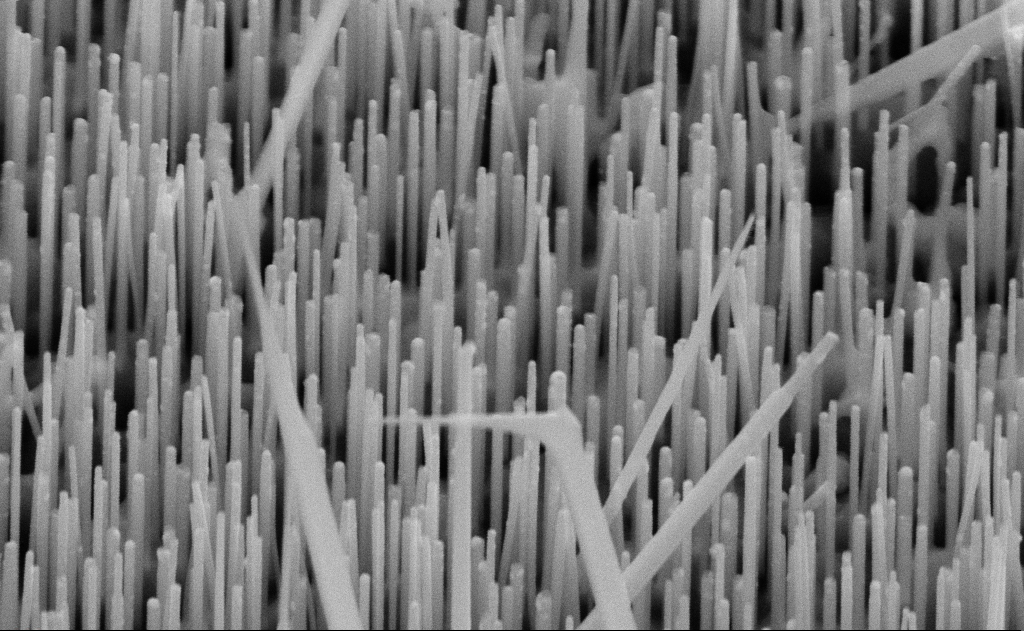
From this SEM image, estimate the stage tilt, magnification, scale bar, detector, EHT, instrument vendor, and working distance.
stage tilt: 45°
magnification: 80 K X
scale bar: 200 nm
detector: SE2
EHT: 10 kV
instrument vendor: Zeiss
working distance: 11 mm